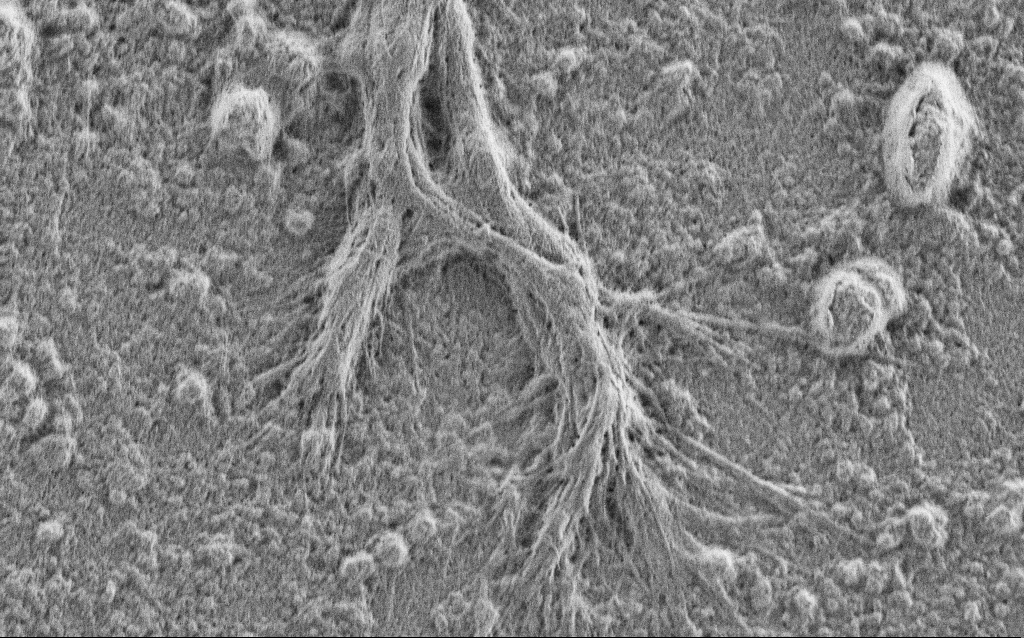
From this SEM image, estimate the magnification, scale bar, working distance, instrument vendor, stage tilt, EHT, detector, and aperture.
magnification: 10 K X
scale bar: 2000 nm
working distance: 4 mm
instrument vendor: Zeiss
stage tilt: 0°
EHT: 0.9 kV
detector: SE2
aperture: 30 µm